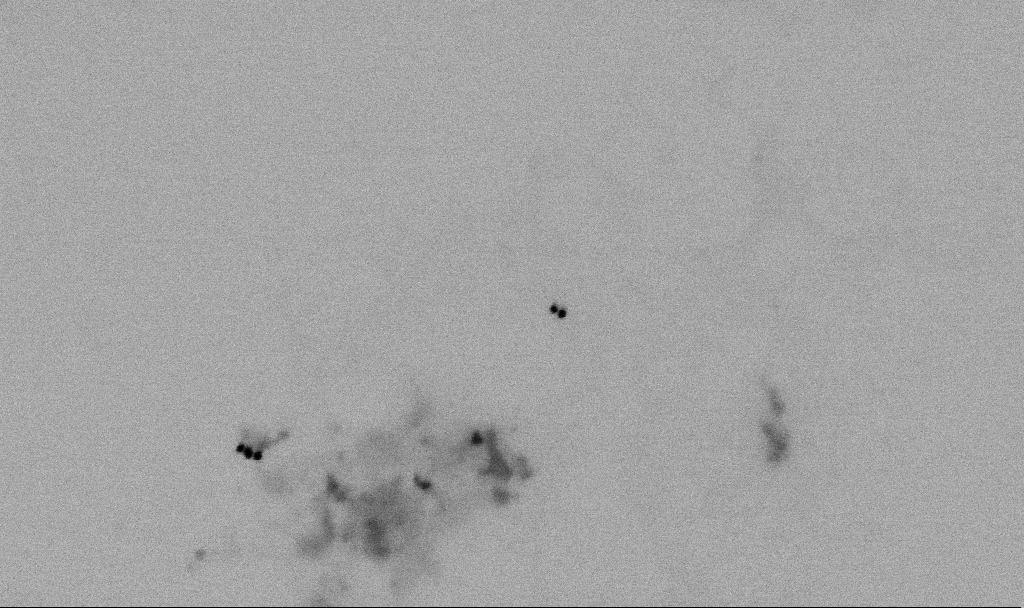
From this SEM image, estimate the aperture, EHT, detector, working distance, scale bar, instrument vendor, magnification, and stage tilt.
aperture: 30 µm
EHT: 2 kV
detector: SE2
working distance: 5.5 mm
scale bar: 100 nm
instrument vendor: Zeiss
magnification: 137.84 K X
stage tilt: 0°